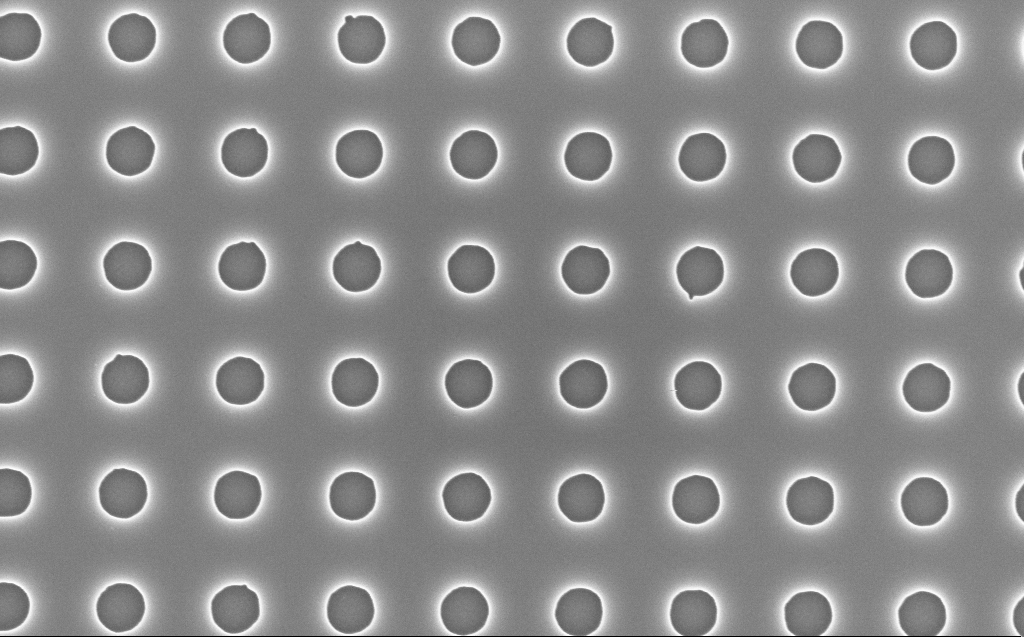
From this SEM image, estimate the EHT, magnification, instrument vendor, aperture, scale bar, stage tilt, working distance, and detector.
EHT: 5 kV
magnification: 40 K X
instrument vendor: Zeiss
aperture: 30 µm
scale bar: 1000 nm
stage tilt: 0°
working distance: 7 mm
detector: InLens